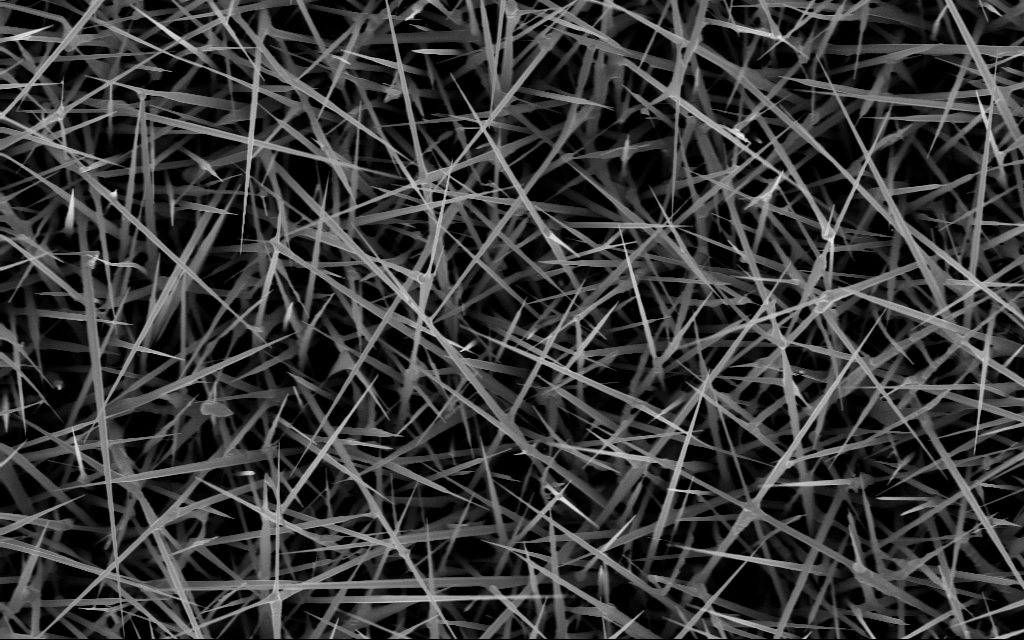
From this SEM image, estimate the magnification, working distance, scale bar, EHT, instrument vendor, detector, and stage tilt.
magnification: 20 K X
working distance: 7 mm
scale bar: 2000 nm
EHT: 10 kV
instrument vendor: Zeiss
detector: InLens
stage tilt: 0°